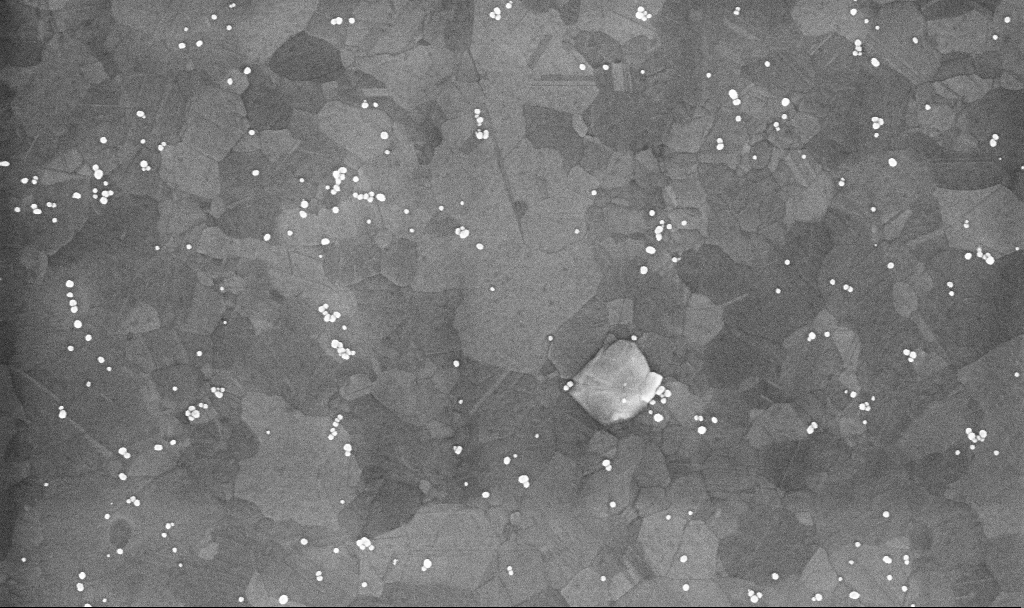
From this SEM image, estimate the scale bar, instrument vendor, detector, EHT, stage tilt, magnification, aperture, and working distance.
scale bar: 200 nm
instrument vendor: Zeiss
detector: InLens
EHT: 10 kV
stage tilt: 0°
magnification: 100.42 K X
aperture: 30 µm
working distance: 3.4 mm